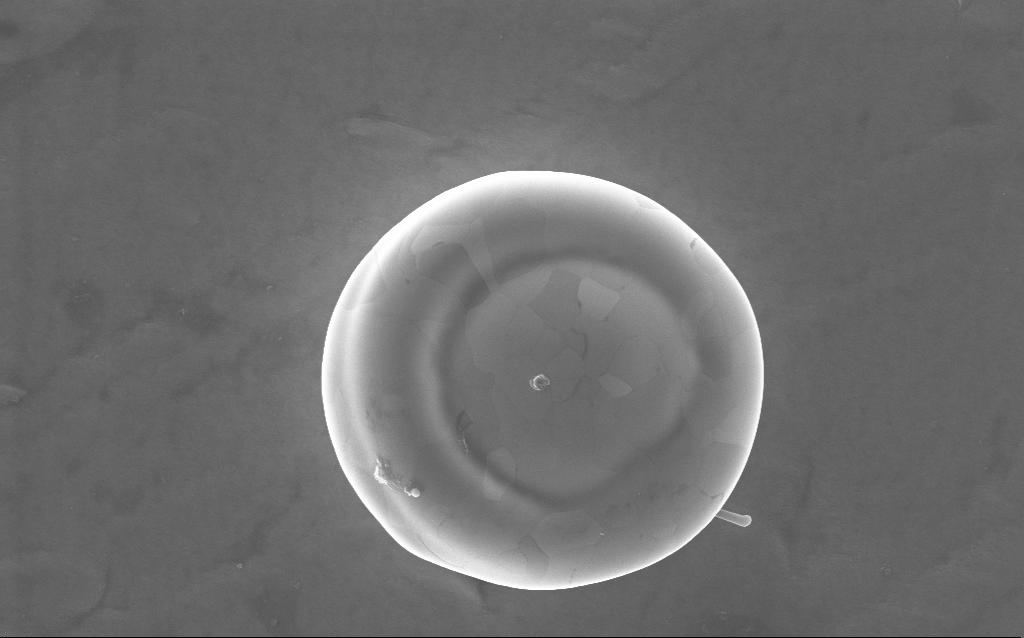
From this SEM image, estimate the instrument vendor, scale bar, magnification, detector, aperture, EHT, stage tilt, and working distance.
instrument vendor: Zeiss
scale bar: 2000 nm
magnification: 36 K X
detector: InLens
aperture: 30 µm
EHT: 10 kV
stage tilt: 0°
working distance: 2 mm